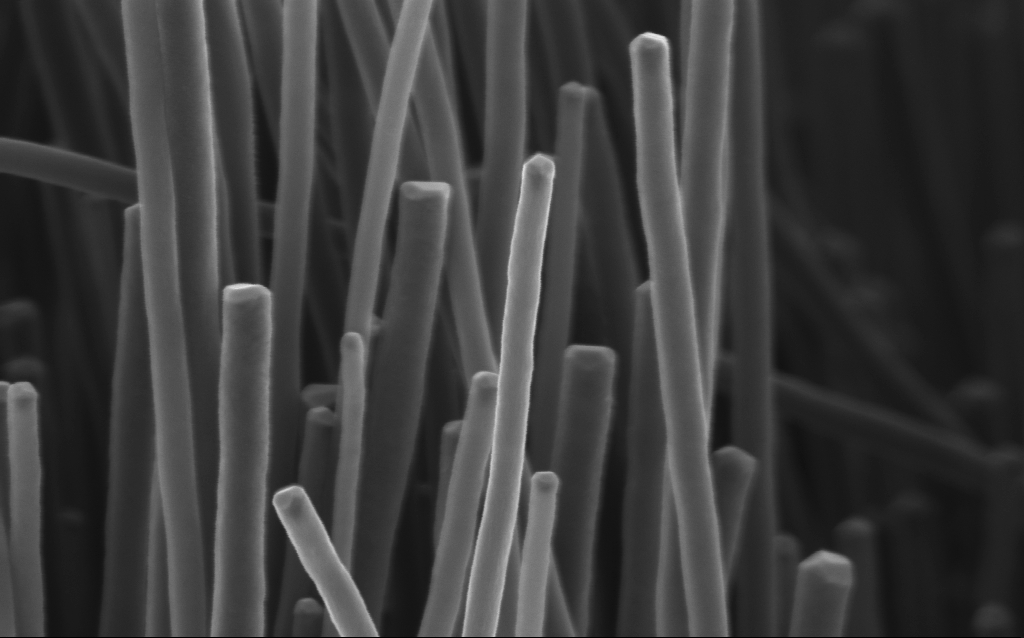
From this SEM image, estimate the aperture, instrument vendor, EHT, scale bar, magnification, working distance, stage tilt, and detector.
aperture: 30 µm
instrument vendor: Zeiss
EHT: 3 kV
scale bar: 200 nm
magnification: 83.35 K X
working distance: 3 mm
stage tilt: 0°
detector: InLens